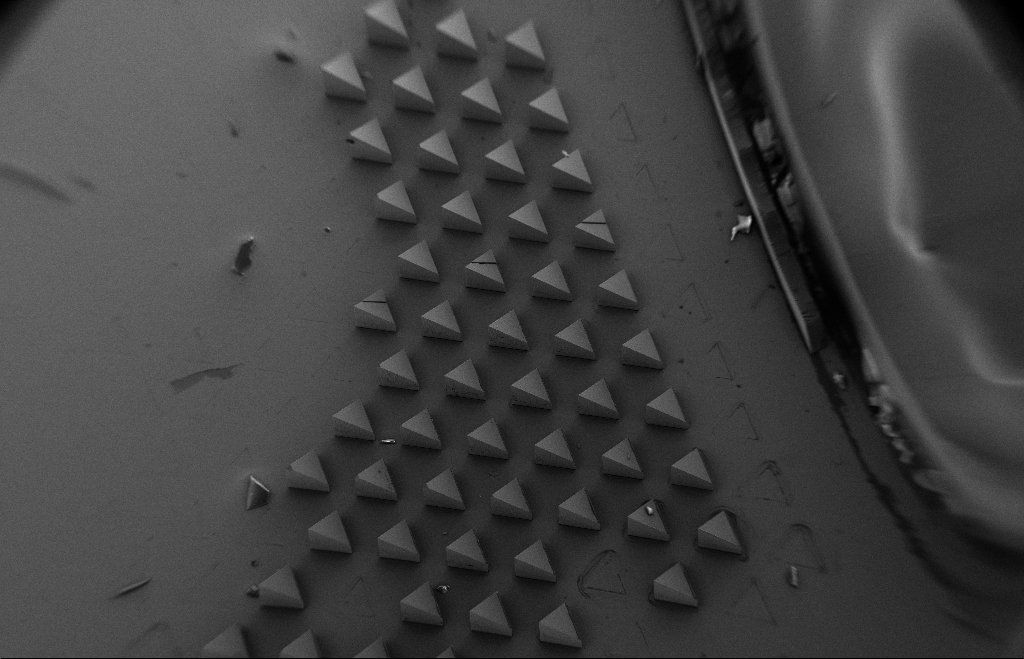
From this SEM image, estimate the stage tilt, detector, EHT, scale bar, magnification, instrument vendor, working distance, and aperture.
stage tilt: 20.1°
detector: SE2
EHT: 5 kV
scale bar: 200000 nm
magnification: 0.054 K X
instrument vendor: Zeiss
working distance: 13 mm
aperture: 30 µm